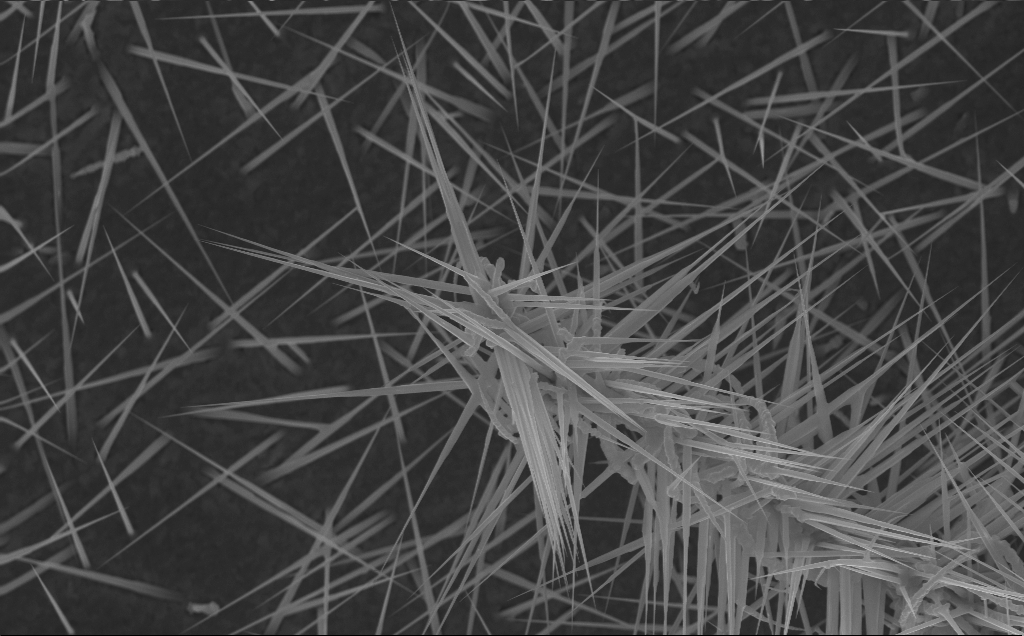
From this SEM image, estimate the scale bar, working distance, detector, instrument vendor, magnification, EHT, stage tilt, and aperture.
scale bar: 2000 nm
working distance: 6 mm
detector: InLens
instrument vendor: Zeiss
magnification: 21.54 K X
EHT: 10 kV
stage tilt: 0°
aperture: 30 µm